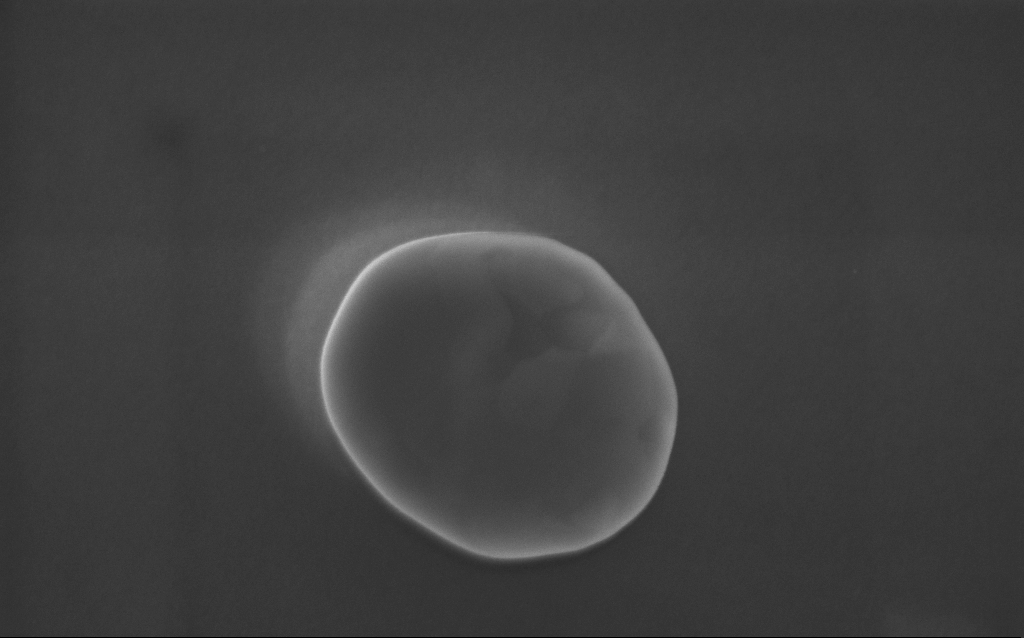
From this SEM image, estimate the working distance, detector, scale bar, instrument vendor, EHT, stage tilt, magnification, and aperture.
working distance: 3 mm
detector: InLens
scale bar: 200 nm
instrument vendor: Zeiss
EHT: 5 kV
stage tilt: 0°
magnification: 141 K X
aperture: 30 µm